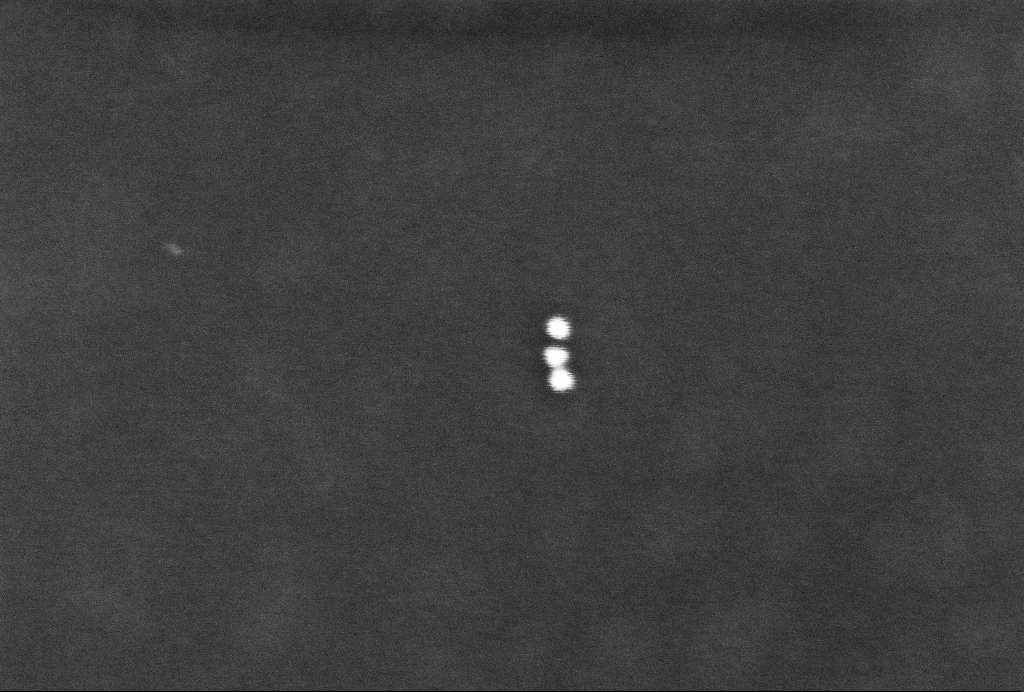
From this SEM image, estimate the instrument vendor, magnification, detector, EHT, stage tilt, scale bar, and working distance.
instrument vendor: Zeiss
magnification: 303.6 K X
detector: InLens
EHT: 2 kV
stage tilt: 0°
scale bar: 100 nm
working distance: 3.3 mm